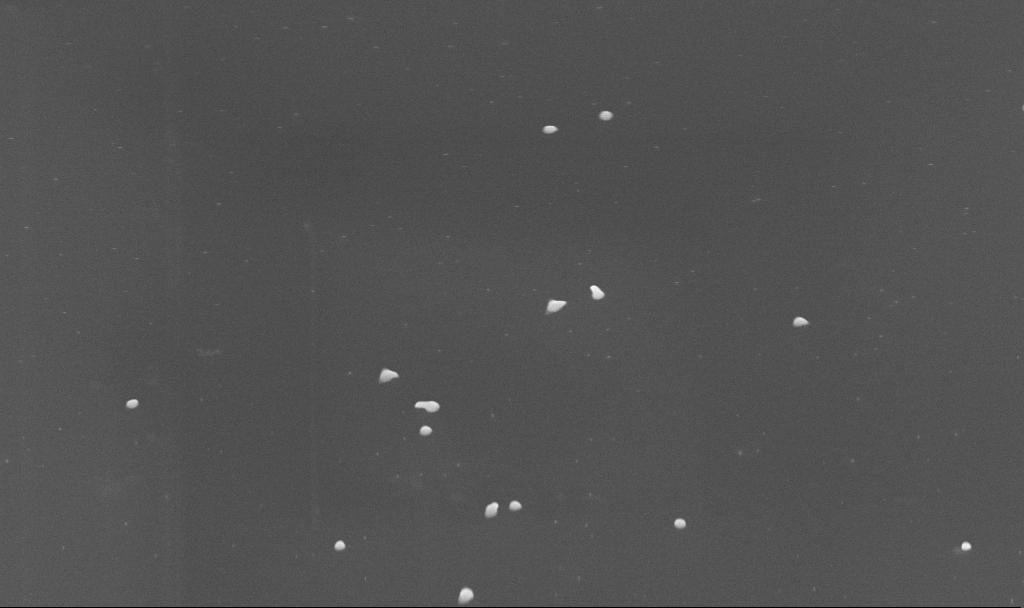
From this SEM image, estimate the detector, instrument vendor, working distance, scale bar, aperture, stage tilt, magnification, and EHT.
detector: InLens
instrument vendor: Zeiss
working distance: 4.7 mm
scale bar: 1000 nm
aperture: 30 µm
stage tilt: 45°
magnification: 50 K X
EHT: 10 kV